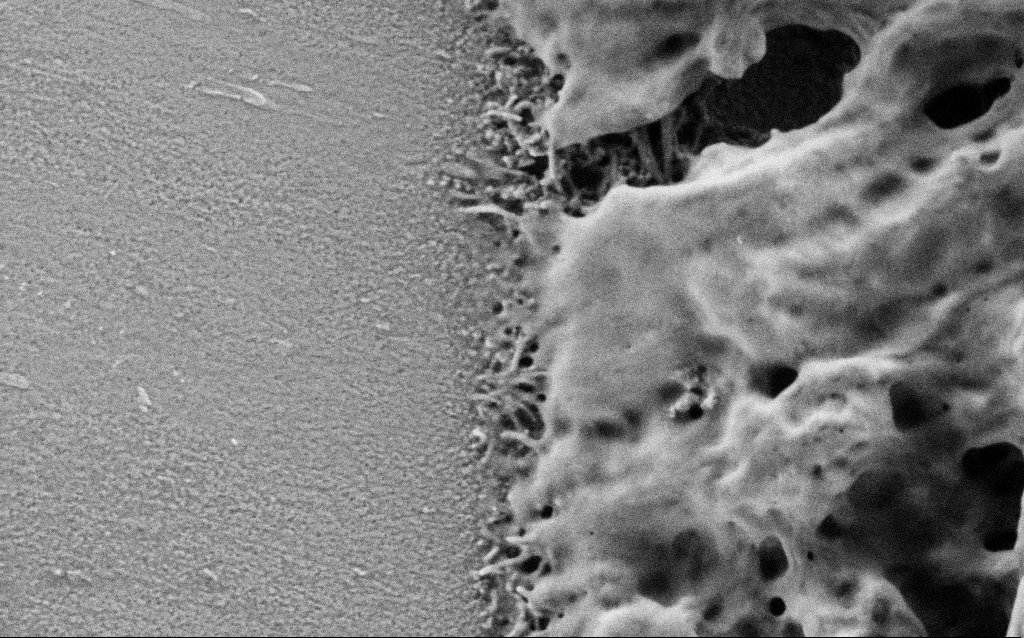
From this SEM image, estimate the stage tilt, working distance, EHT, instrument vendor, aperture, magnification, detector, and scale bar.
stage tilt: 0°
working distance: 4 mm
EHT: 1 kV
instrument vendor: Zeiss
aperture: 30 µm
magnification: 75 K X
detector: SE2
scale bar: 200 nm